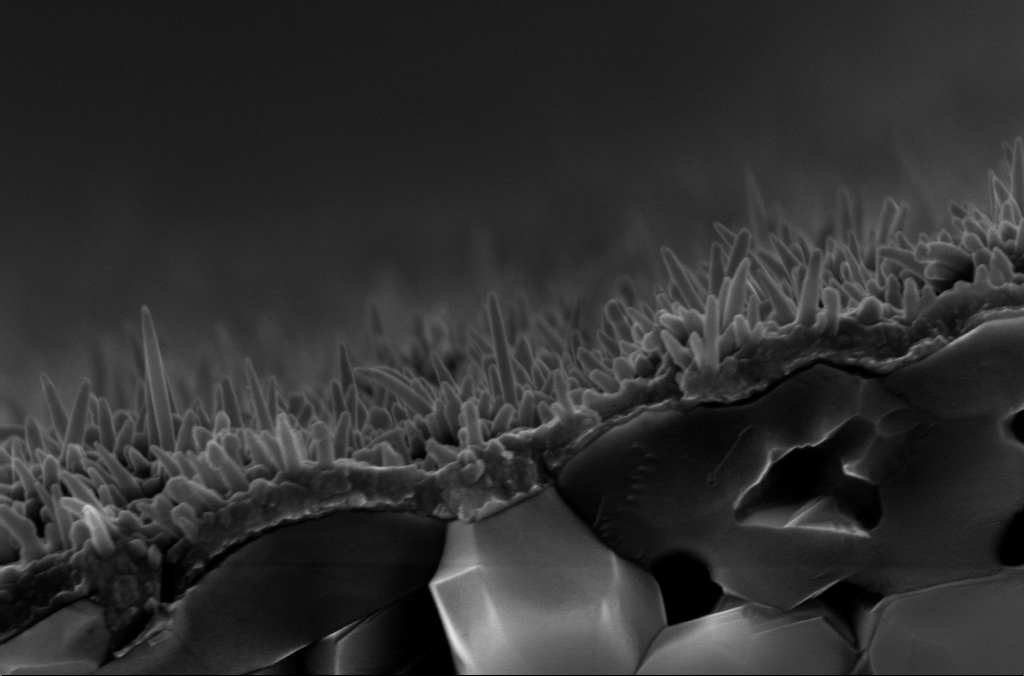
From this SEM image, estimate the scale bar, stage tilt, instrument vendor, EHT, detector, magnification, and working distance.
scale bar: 200 nm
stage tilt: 4°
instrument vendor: Zeiss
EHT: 10 kV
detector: InLens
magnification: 97.08 K X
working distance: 2.6 mm